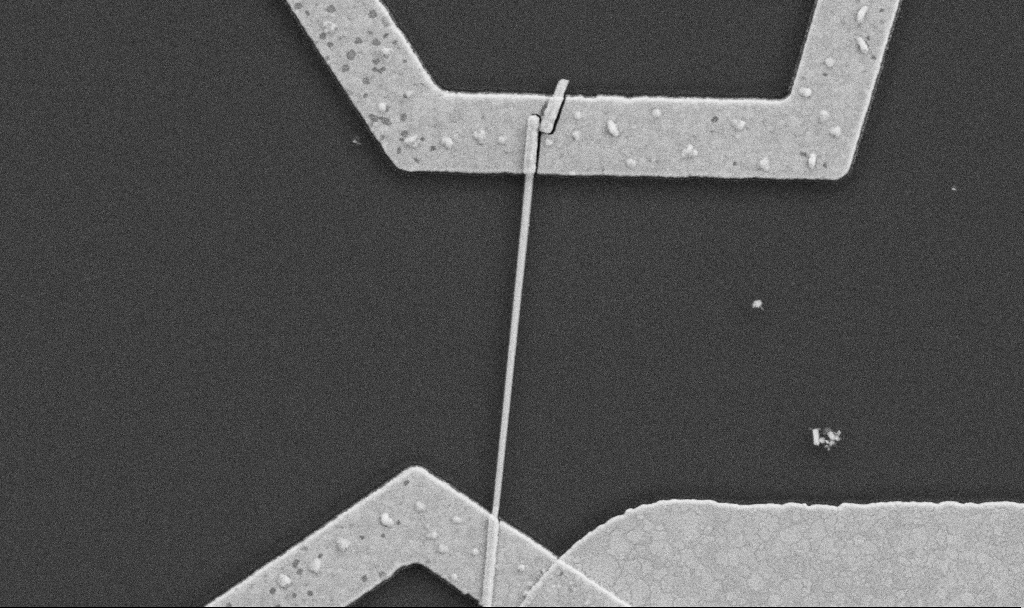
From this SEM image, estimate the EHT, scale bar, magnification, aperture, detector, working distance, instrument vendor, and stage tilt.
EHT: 5 kV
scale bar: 1000 nm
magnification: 30 K X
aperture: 30 µm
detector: SE2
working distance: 8.7 mm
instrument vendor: Zeiss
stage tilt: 0°